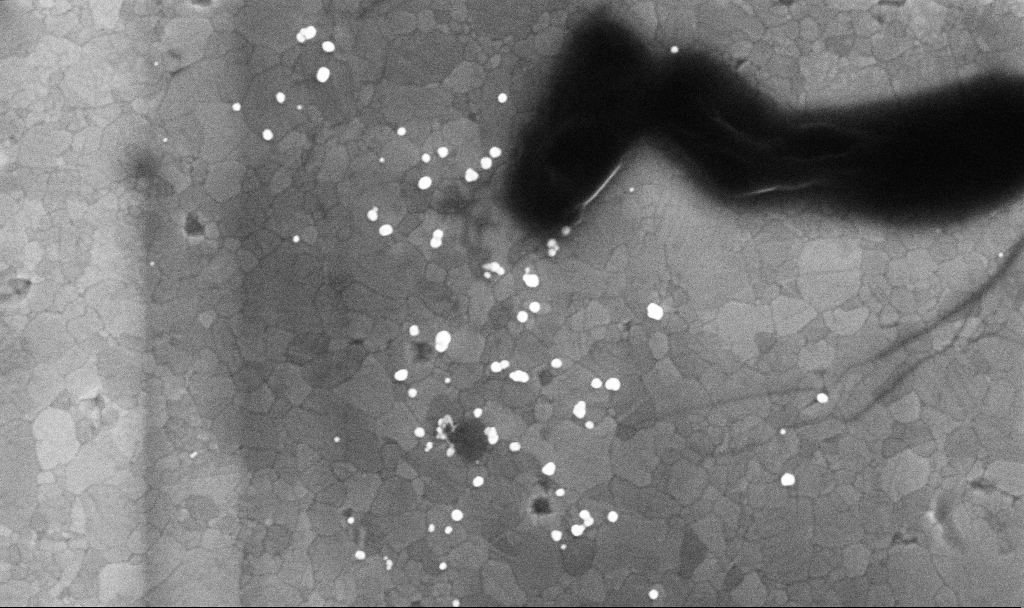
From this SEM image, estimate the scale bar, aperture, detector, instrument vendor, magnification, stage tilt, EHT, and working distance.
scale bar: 1000 nm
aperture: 30 µm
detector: InLens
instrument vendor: Zeiss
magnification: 68.45 K X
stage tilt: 0°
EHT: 10 kV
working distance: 3.4 mm